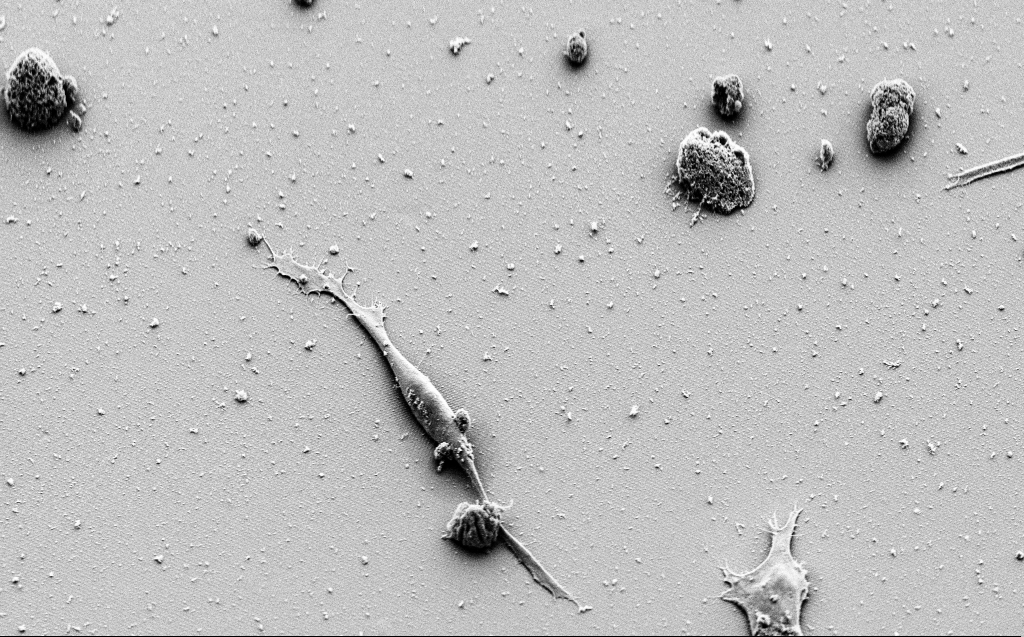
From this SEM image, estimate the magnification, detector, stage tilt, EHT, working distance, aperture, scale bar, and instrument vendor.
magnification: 2.56 K X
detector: SE2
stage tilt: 45°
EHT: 3 kV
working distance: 9 mm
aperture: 30 µm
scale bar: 20000 nm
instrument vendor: Zeiss